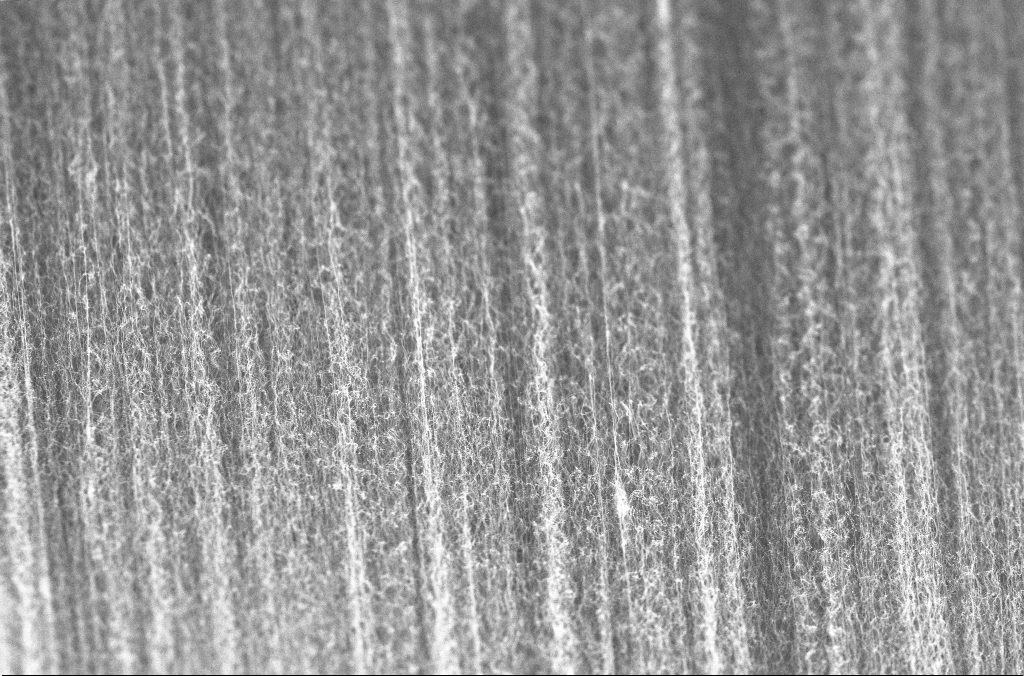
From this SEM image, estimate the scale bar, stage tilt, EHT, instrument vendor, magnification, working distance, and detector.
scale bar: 2000 nm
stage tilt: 0°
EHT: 20 kV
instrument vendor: Zeiss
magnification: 10 K X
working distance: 4 mm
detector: InLens